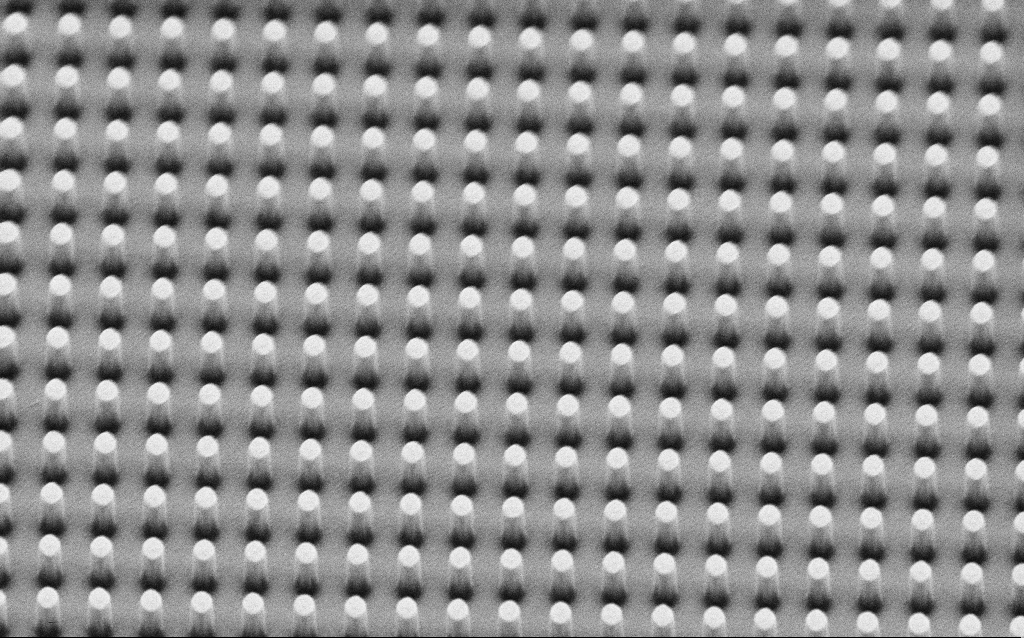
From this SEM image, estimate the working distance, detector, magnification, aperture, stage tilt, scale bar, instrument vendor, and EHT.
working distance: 4.7 mm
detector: SE2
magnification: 38.08 K X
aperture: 30 µm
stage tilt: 45°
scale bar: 1000 nm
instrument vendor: Zeiss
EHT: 3 kV